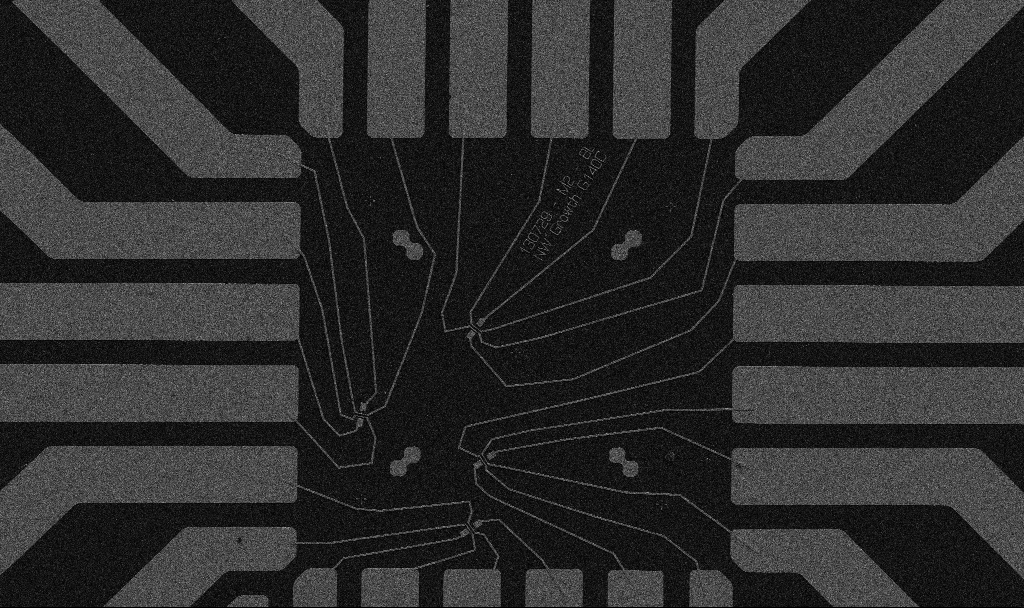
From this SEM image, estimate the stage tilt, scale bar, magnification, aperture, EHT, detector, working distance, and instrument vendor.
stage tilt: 0°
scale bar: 20000 nm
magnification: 1 K X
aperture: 30 µm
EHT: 5 kV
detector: SE2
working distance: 10.7 mm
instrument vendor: Zeiss